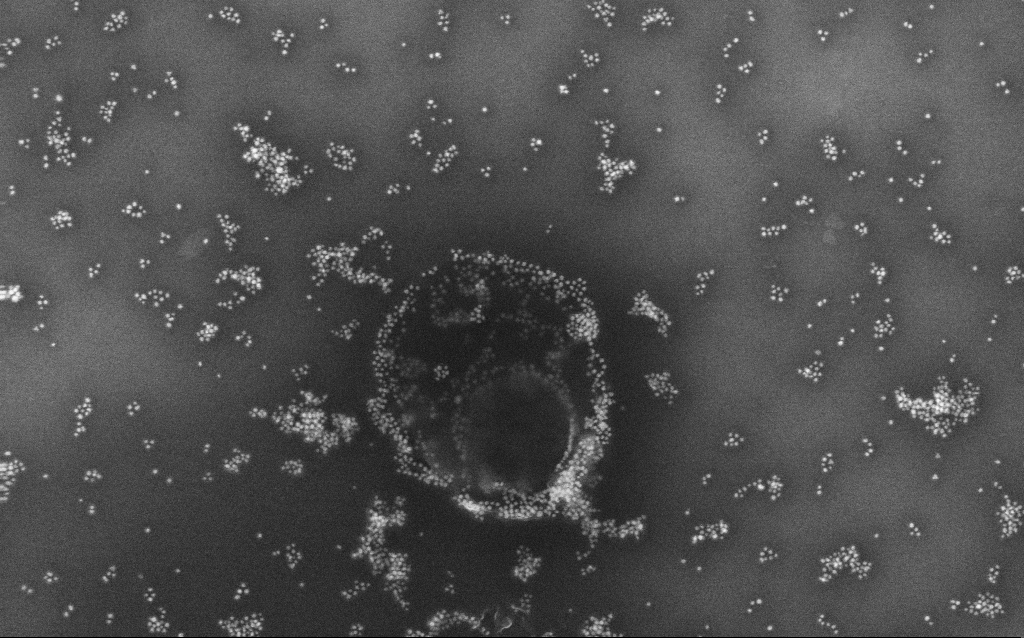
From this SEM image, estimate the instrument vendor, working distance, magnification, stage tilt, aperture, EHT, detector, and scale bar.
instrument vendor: Zeiss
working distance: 1.8 mm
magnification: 100 K X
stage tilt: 0°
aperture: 30 µm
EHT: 10 kV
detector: InLens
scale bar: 200 nm